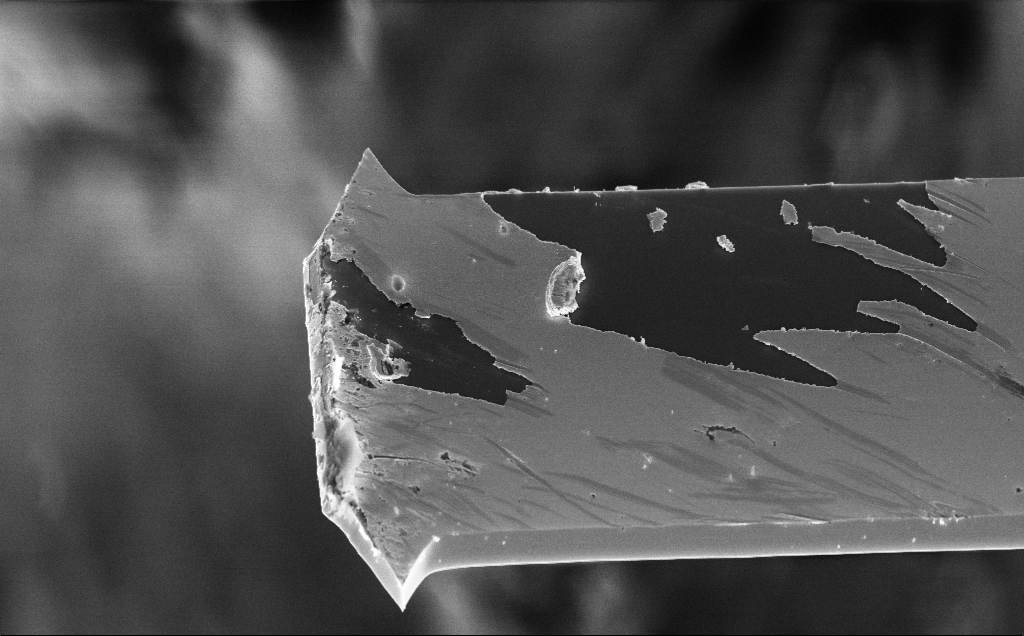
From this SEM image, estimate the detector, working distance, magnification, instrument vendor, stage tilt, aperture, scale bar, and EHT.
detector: InLens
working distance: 3 mm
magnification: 3.72 K X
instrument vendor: Zeiss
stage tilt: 44.2°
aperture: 30 µm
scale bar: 10000 nm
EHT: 10 kV